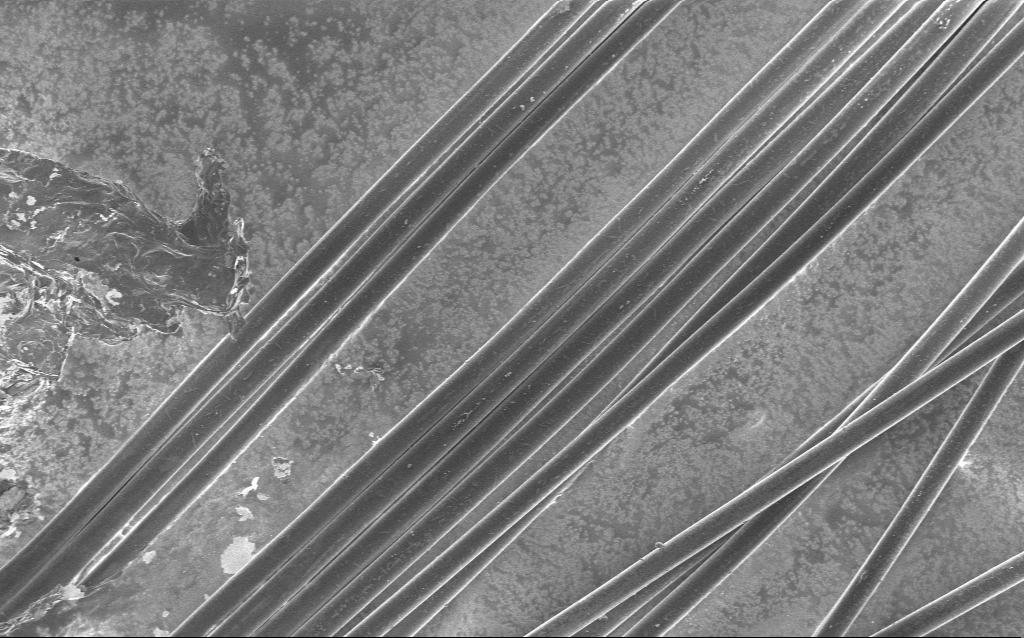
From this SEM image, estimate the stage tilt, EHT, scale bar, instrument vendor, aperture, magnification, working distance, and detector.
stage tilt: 0°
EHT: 1 kV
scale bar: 20000 nm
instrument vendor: Zeiss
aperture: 30 µm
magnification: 0.73 K X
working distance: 5 mm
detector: InLens